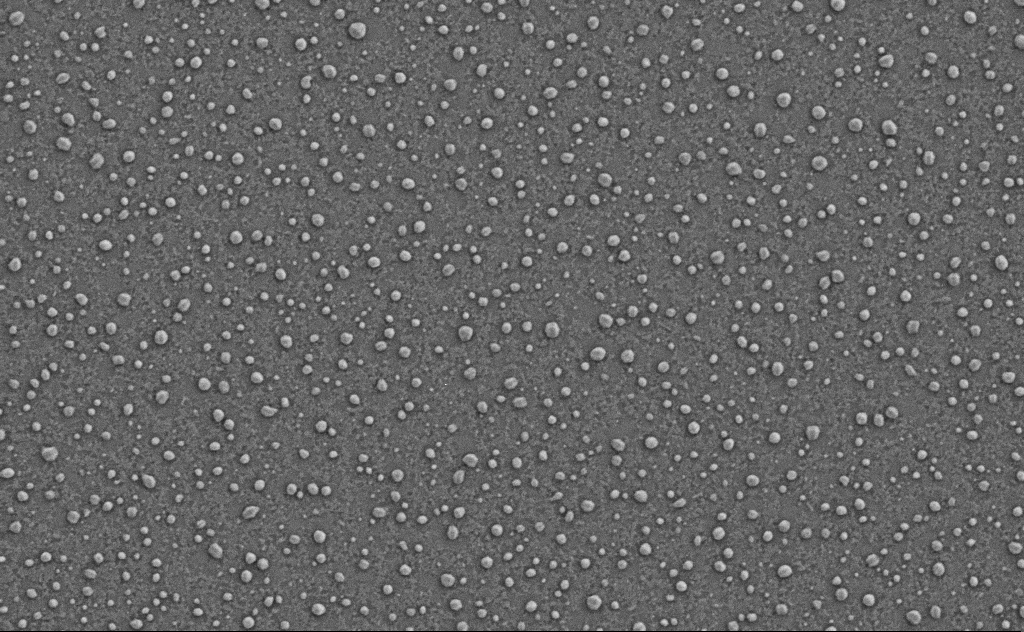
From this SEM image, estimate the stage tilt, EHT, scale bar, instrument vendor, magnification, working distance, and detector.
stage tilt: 0°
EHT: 3 kV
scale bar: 1000 nm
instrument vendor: Zeiss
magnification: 40 K X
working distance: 4 mm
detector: SE2